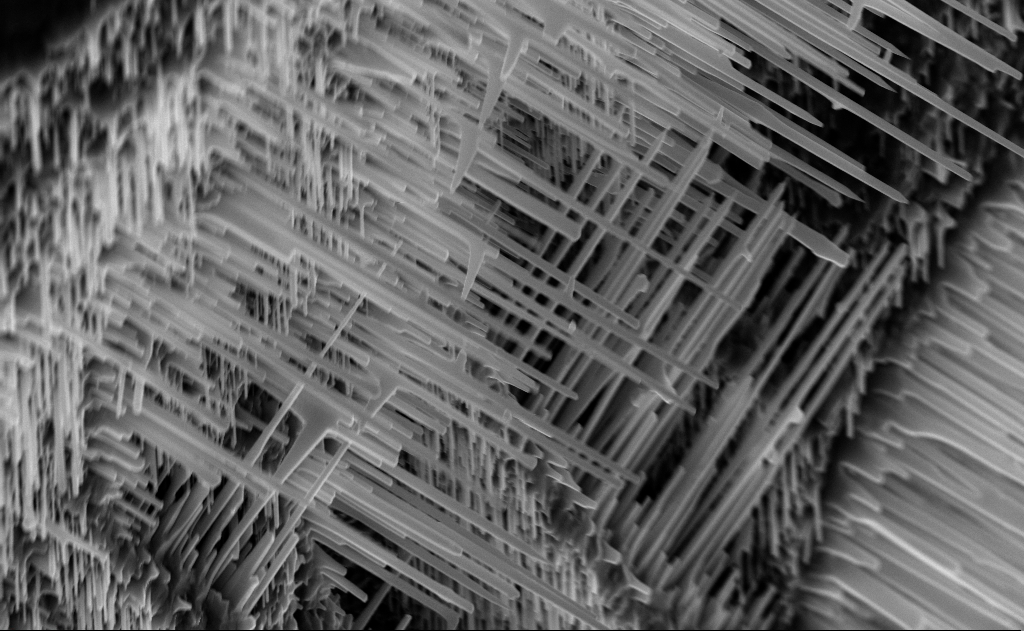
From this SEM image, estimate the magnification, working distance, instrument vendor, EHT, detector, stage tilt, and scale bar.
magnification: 20 K X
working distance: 6 mm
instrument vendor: Zeiss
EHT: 10 kV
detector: InLens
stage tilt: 0°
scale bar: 2000 nm